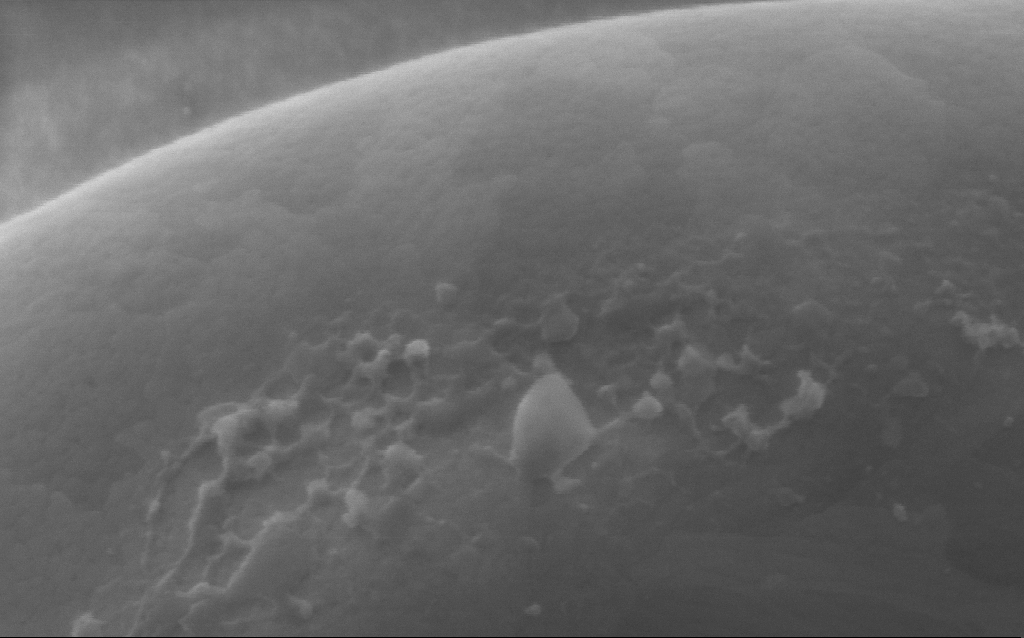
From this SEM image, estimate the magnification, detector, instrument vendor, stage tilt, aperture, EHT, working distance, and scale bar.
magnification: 254 K X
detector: InLens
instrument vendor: Zeiss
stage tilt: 0°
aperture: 30 µm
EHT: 5 kV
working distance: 4 mm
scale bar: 200 nm